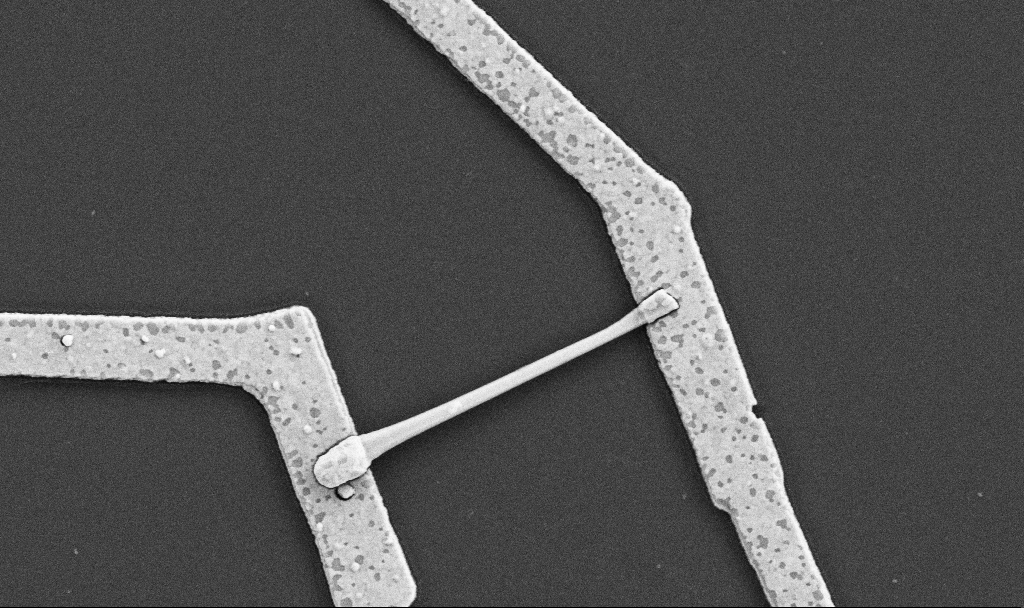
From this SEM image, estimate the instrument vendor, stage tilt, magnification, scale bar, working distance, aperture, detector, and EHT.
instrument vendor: Zeiss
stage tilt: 0°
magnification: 30 K X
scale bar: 1000 nm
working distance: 8.7 mm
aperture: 30 µm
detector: SE2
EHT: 5 kV